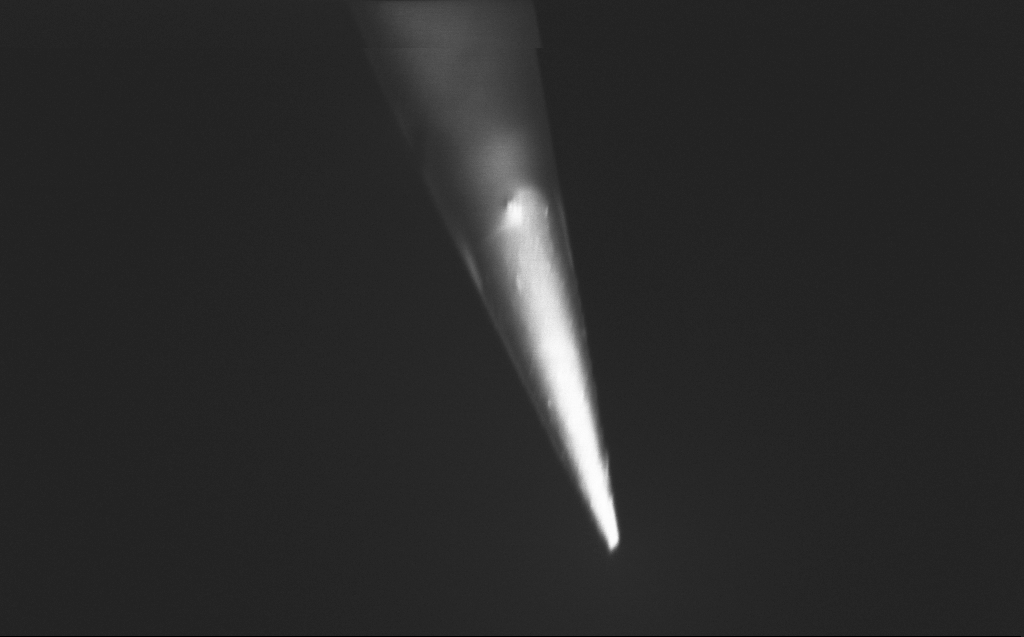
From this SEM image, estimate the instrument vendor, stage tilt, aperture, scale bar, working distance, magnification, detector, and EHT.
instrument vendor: Zeiss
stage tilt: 39.3°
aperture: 30 µm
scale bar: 1000 nm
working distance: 5 mm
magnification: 50 K X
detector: InLens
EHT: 0.8 kV